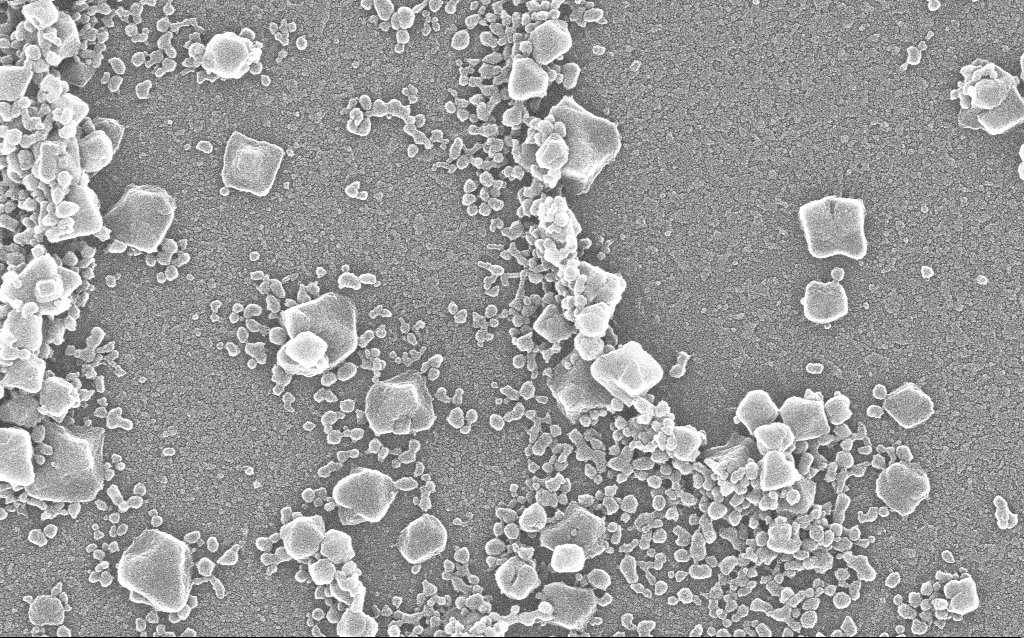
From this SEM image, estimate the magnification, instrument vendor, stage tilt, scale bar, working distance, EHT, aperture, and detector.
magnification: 100 K X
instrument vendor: Zeiss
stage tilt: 0°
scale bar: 200 nm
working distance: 1.9 mm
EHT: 20 kV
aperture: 30 µm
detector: InLens